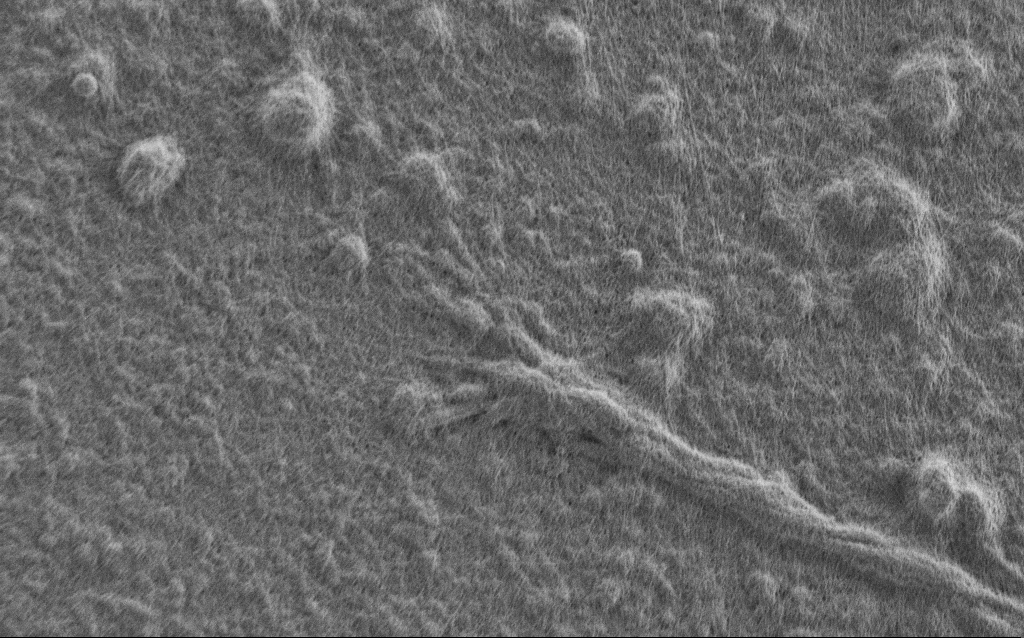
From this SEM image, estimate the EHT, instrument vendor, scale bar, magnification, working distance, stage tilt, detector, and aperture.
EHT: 0.9 kV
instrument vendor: Zeiss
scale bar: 2000 nm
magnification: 10 K X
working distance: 7 mm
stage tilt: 0°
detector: SE2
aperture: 30 µm